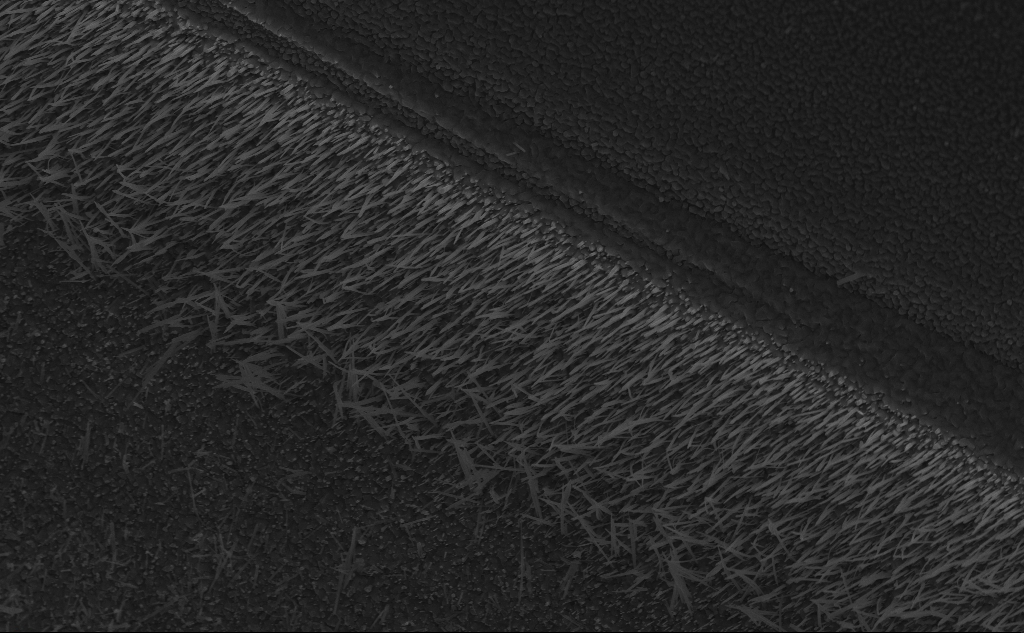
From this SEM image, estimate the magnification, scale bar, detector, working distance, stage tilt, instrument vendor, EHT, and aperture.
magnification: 14.81 K X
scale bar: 2000 nm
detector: InLens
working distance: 6 mm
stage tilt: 45°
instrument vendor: Zeiss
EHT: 10 kV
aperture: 30 µm